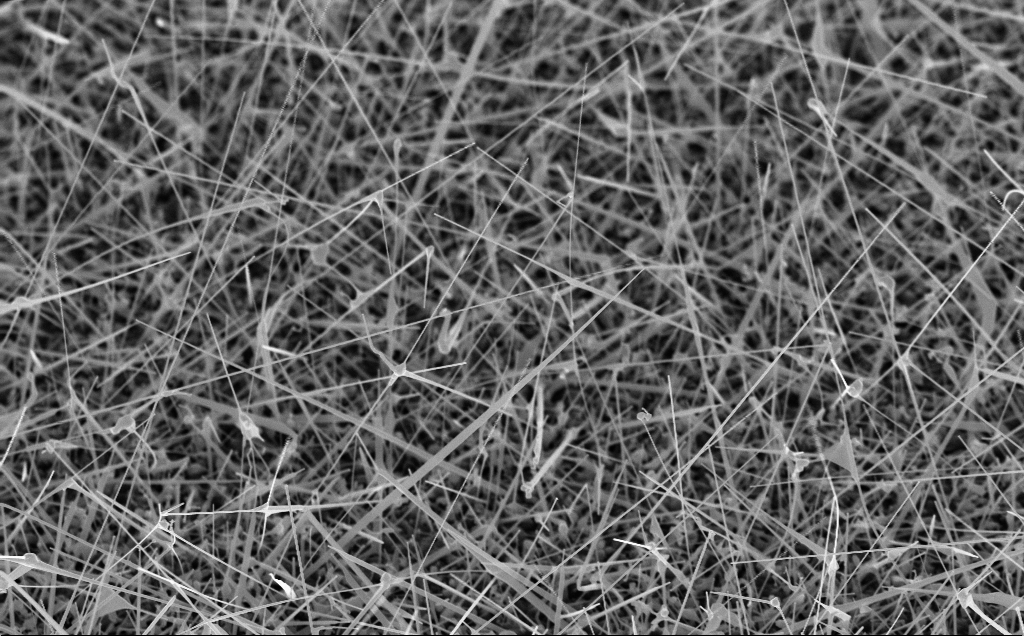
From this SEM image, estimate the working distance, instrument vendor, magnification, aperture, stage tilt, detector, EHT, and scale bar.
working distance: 8 mm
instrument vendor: Zeiss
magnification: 20.35 K X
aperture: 30 µm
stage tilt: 45°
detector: InLens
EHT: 10 kV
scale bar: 1000 nm